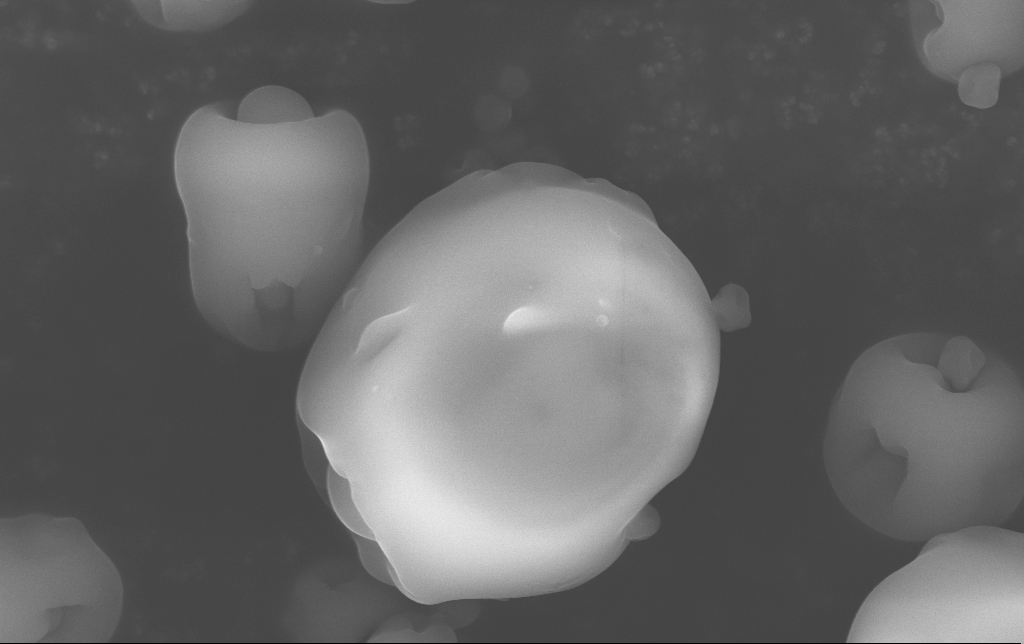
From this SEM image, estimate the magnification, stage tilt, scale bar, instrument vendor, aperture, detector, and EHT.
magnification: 32.54 K X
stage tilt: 0°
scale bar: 1000 nm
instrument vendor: Zeiss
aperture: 30 µm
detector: InLens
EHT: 15 kV